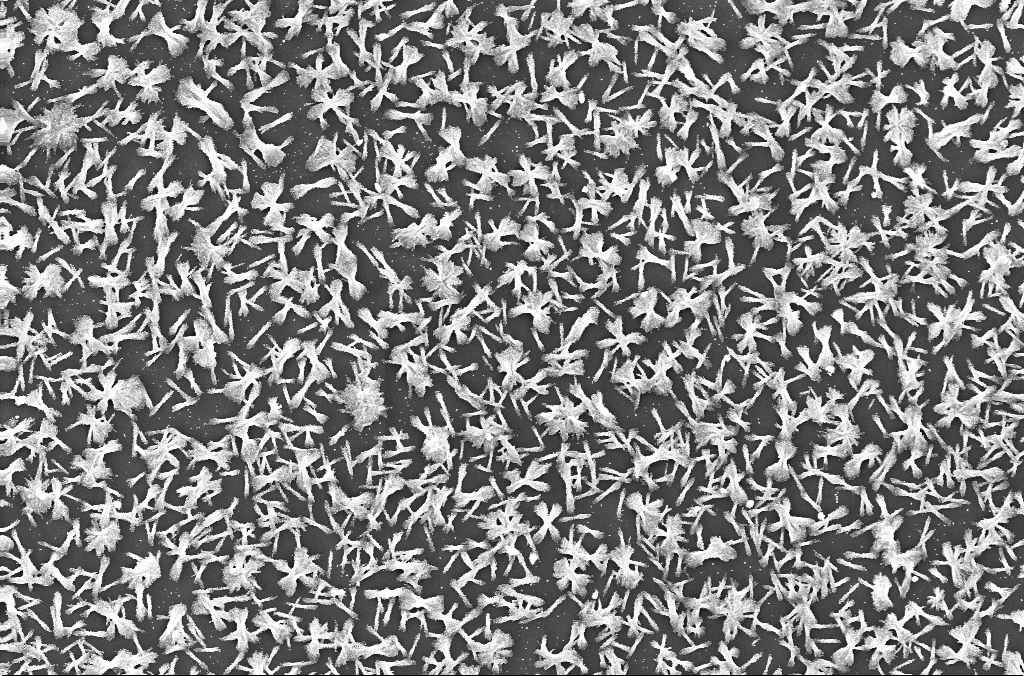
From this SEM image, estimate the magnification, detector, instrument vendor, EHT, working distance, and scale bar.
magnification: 2 K X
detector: InLens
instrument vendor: Zeiss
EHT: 20 kV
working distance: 2.8 mm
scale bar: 10000 nm